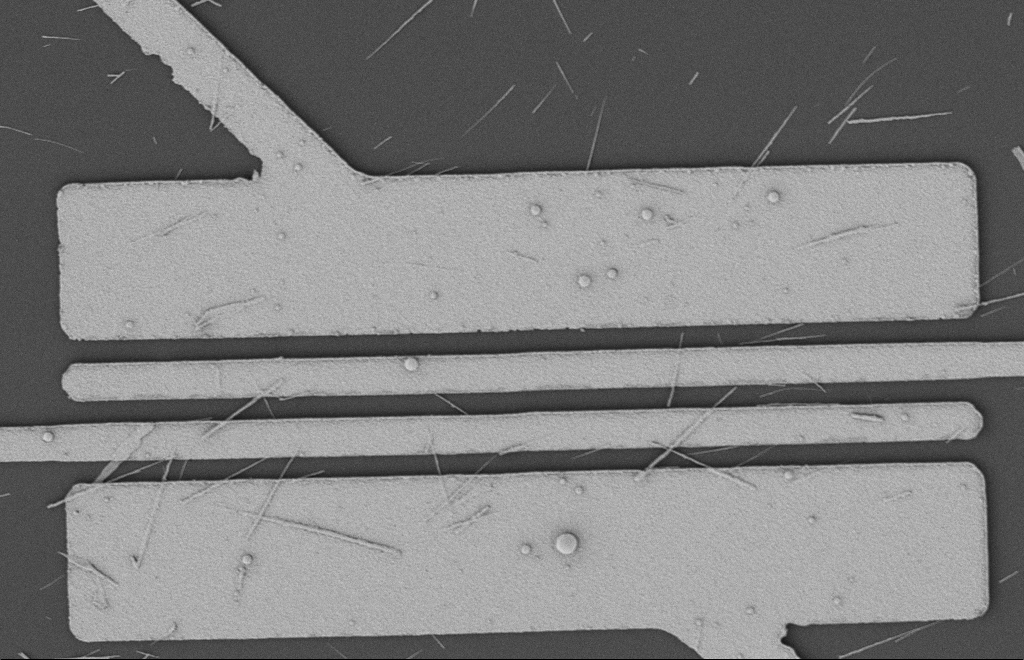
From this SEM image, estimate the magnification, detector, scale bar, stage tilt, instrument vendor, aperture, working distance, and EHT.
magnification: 5.5 K X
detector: SE2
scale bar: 2000 nm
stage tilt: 0°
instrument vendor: Zeiss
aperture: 20 µm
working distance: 12 mm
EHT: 2 kV